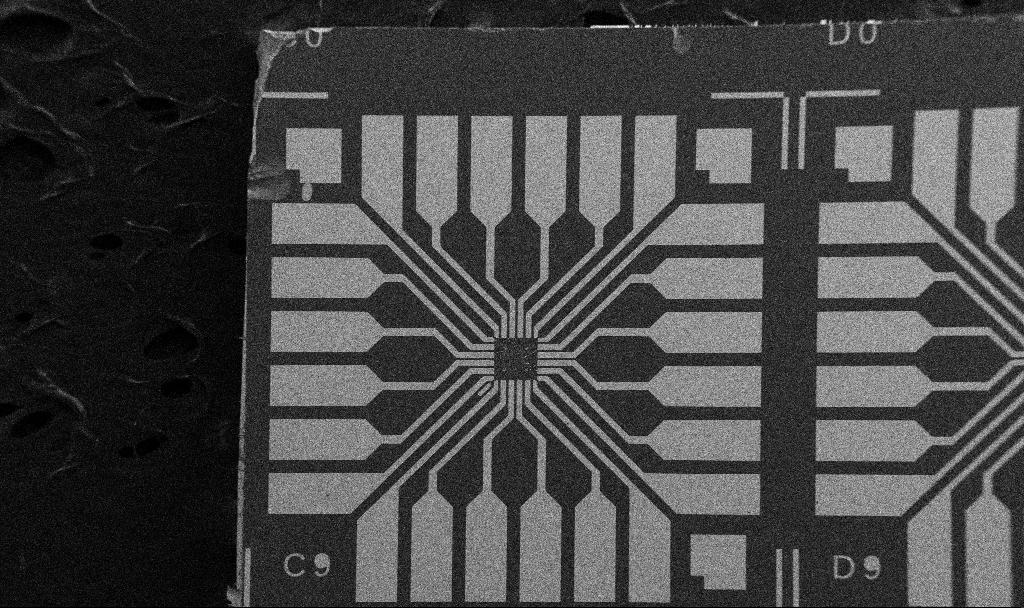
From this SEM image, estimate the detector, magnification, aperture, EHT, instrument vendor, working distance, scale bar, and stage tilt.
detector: SE2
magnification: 0.1 K X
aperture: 30 µm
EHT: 5 kV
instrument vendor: Zeiss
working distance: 10.7 mm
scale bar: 200000 nm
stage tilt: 0°